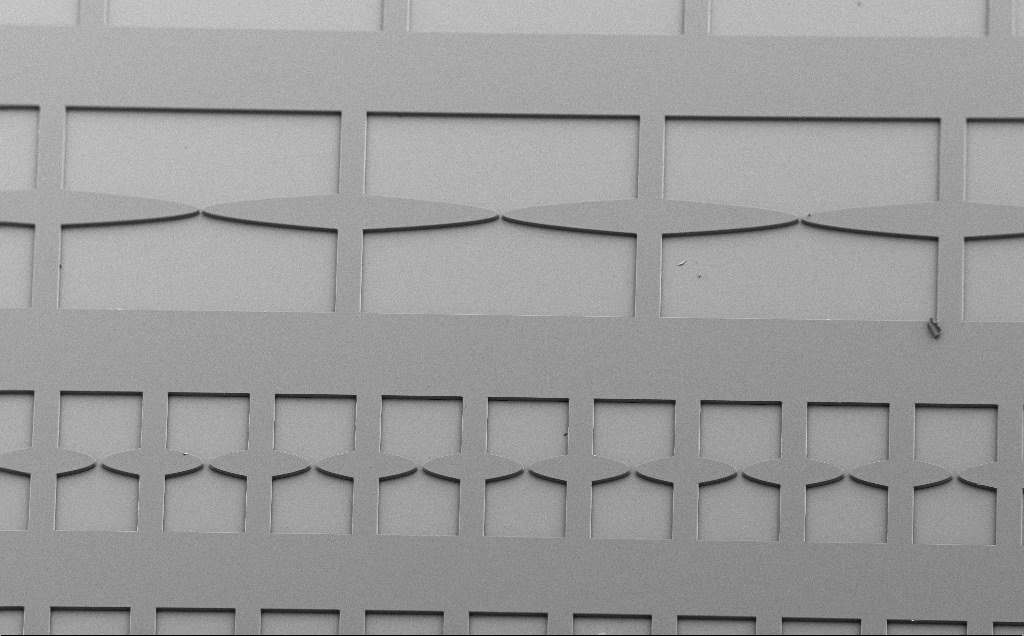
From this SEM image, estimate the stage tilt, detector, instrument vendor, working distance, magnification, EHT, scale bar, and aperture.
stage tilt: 40°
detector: SE2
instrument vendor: Zeiss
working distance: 8 mm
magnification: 0.187 K X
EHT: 5 kV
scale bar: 100000 nm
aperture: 30 µm